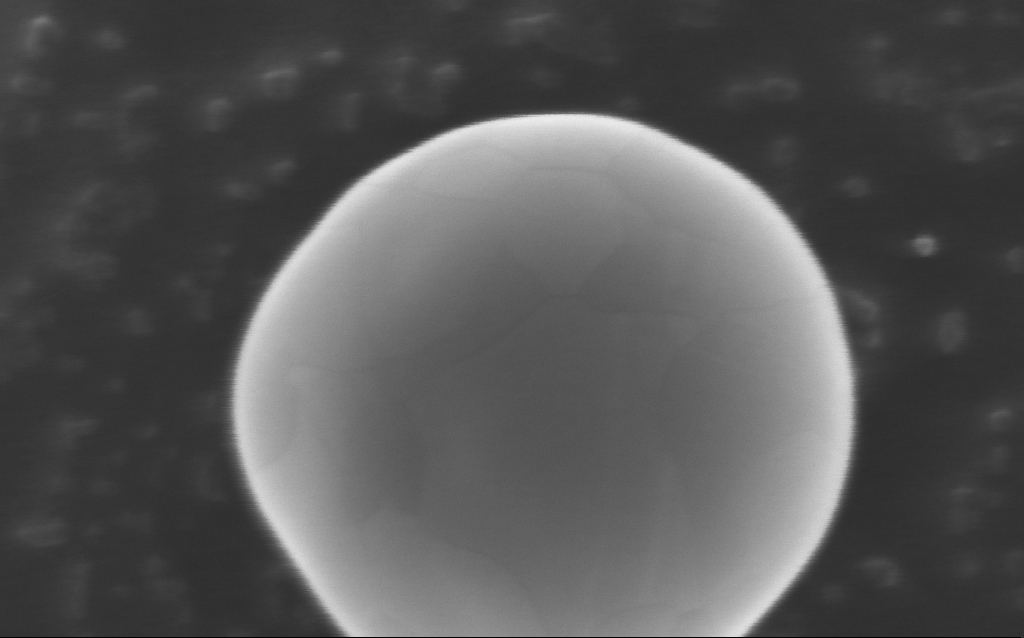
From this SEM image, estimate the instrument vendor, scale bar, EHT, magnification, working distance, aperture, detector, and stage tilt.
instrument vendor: Zeiss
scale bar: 100 nm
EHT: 10 kV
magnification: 489.75 K X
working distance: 5 mm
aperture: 30 µm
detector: InLens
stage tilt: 0°